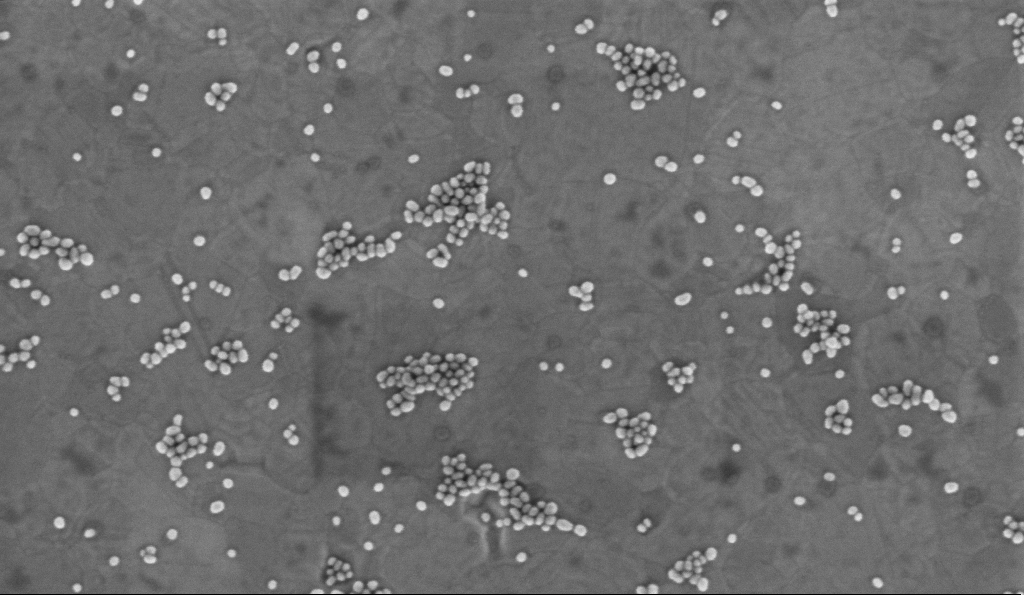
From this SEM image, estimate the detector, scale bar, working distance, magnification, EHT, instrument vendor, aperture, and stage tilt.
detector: InLens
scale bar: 100 nm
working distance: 3.2 mm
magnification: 150 K X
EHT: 2 kV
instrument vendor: Zeiss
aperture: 30 µm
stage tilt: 0°